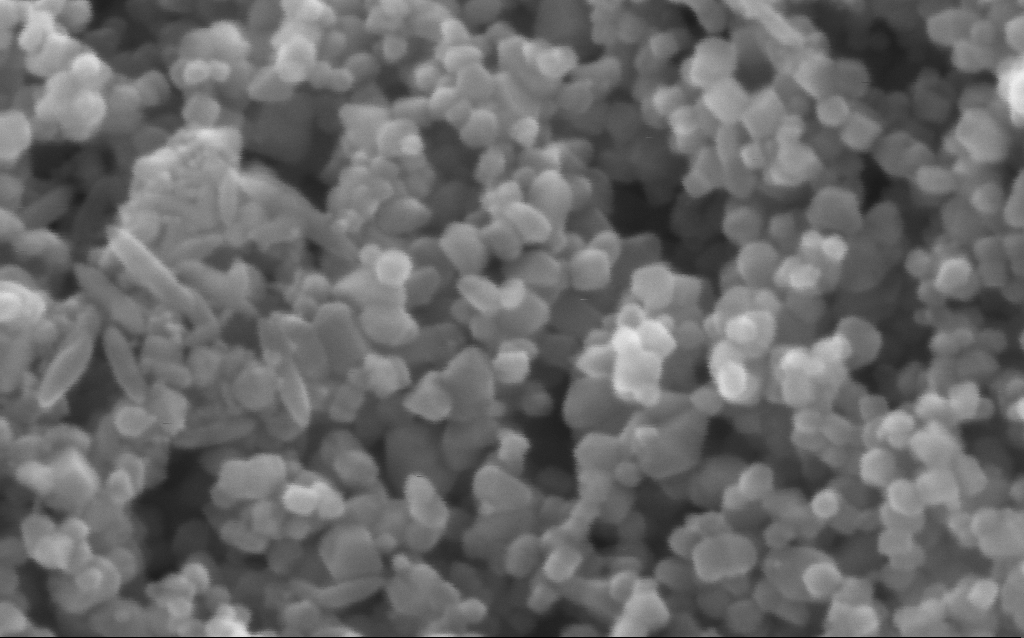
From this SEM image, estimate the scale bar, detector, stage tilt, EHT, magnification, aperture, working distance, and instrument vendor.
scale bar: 100 nm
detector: InLens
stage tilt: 0°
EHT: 5 kV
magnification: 600 K X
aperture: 30 µm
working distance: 4.4 mm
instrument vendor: Zeiss